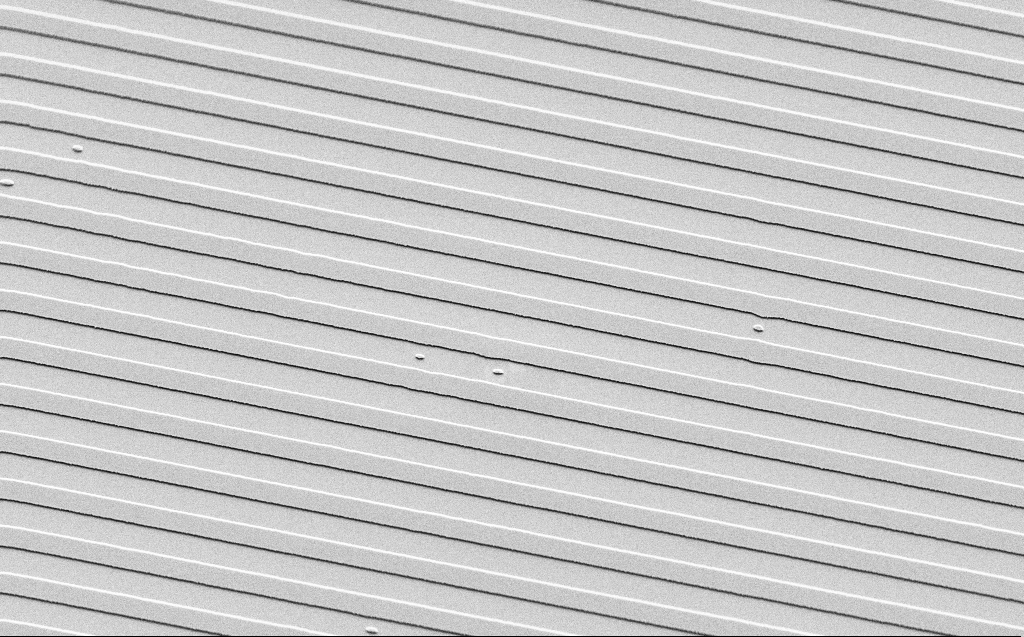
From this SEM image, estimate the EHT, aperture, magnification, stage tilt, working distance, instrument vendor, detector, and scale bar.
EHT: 3 kV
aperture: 30 µm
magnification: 4.29 K X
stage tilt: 45°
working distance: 9 mm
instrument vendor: Zeiss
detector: SE2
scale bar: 10000 nm